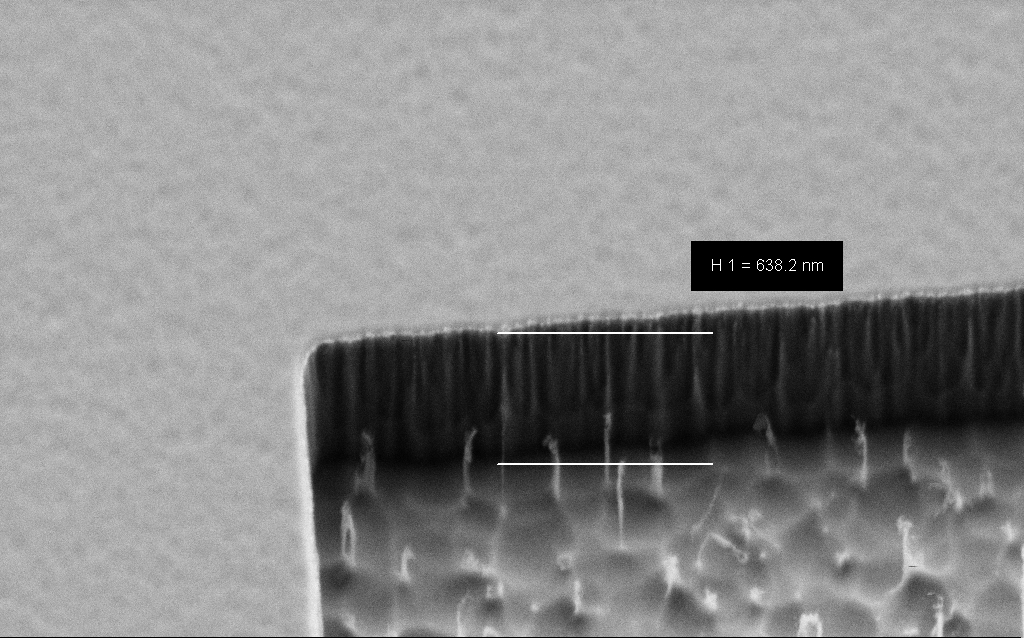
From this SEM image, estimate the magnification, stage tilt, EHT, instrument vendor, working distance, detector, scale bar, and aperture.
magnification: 75.37 K X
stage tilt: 45°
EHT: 2 kV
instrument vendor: Zeiss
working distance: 6 mm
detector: SE2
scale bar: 200 nm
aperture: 30 µm